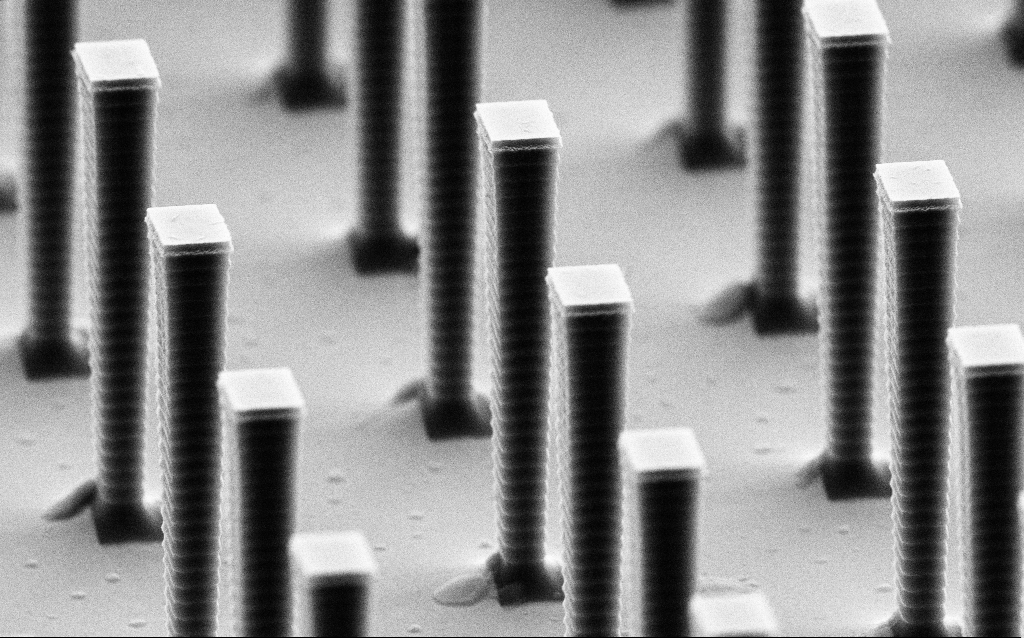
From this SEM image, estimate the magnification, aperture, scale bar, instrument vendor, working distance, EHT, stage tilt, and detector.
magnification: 15 K X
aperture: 30 µm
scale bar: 2000 nm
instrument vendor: Zeiss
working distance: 6.5 mm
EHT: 8 kV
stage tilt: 70°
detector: SE2